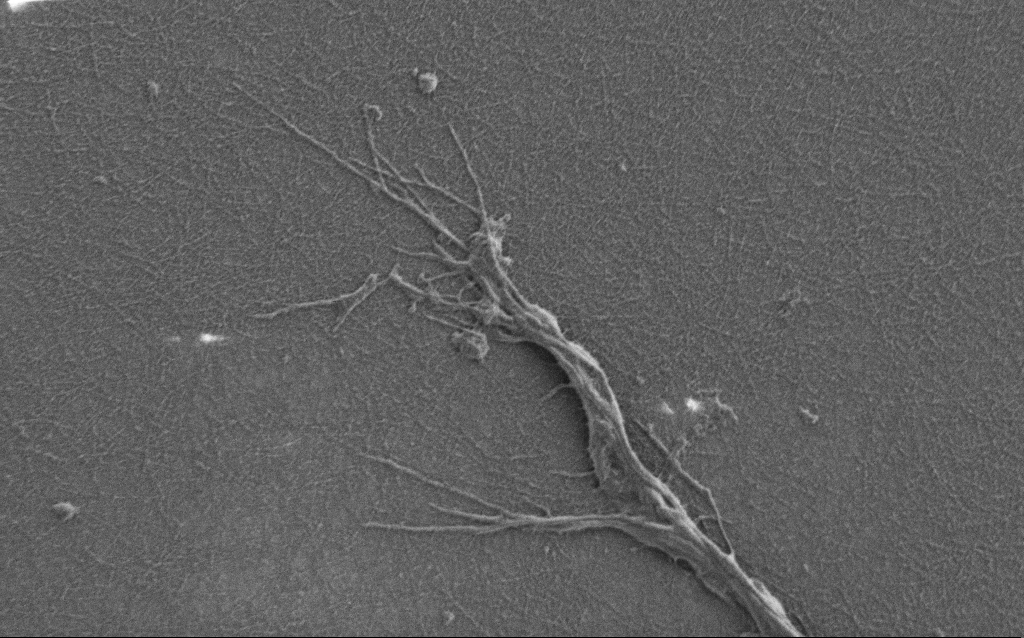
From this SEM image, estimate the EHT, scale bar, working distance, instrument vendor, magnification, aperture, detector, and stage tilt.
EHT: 0.9 kV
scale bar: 2000 nm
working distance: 7 mm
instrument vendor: Zeiss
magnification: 10 K X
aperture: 30 µm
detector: SE2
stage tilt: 0°